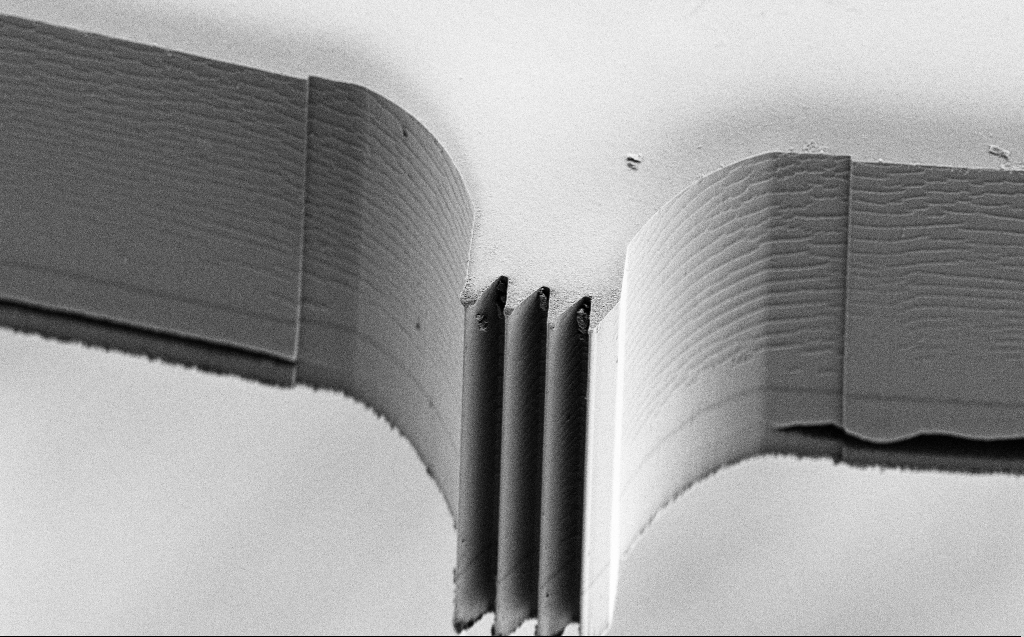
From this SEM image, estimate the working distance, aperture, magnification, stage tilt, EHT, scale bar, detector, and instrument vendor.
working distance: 6 mm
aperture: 30 µm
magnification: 1.37 K X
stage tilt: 45°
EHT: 5 kV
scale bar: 10000 nm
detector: SE2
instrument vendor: Zeiss